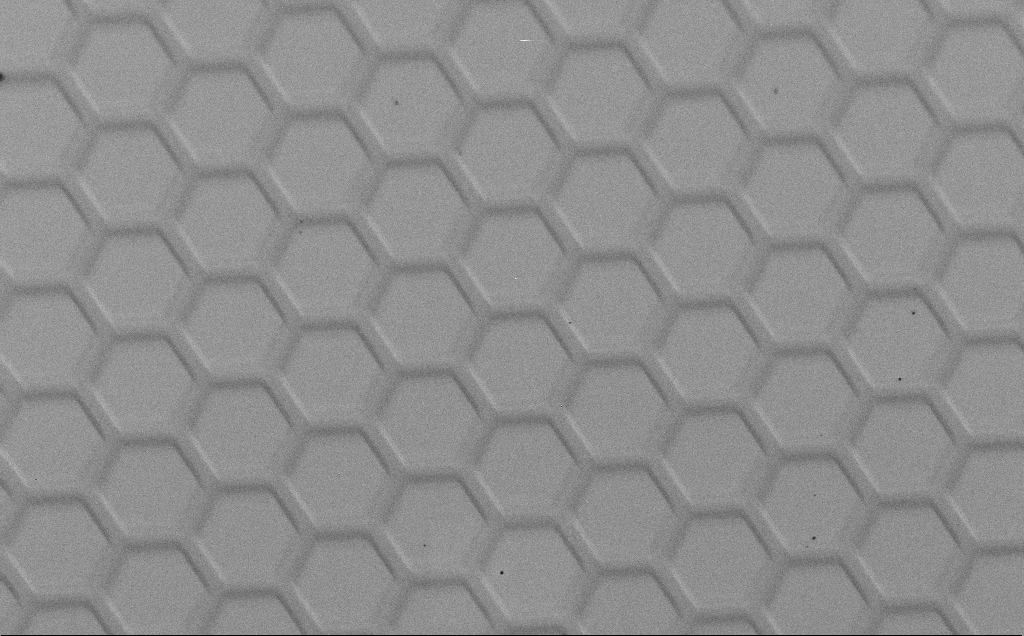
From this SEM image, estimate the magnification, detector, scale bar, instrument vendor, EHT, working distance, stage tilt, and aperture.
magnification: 0.675 K X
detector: SE2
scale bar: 100000 nm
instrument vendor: Zeiss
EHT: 1.5 kV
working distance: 4 mm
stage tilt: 45°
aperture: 30 µm